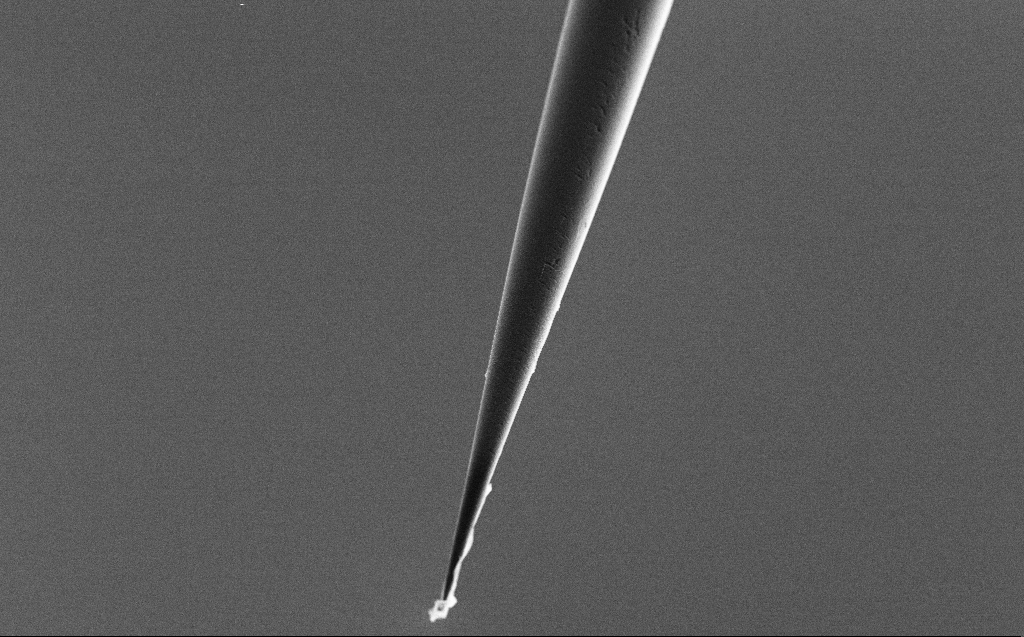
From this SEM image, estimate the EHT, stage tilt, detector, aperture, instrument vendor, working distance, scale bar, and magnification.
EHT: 5 kV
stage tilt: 45°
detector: SE2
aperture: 30 µm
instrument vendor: Zeiss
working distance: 6 mm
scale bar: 10000 nm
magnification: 2.5 K X